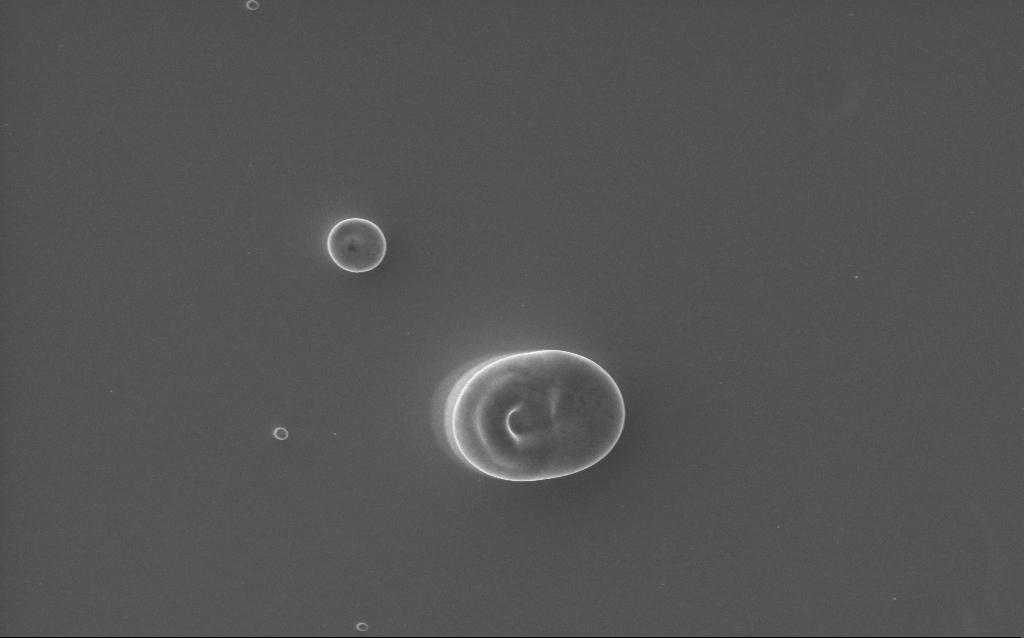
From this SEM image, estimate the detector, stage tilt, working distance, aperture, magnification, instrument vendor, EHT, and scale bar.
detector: InLens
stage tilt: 0°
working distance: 2 mm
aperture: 30 µm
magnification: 12.43 K X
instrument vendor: Zeiss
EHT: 5 kV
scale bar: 2000 nm